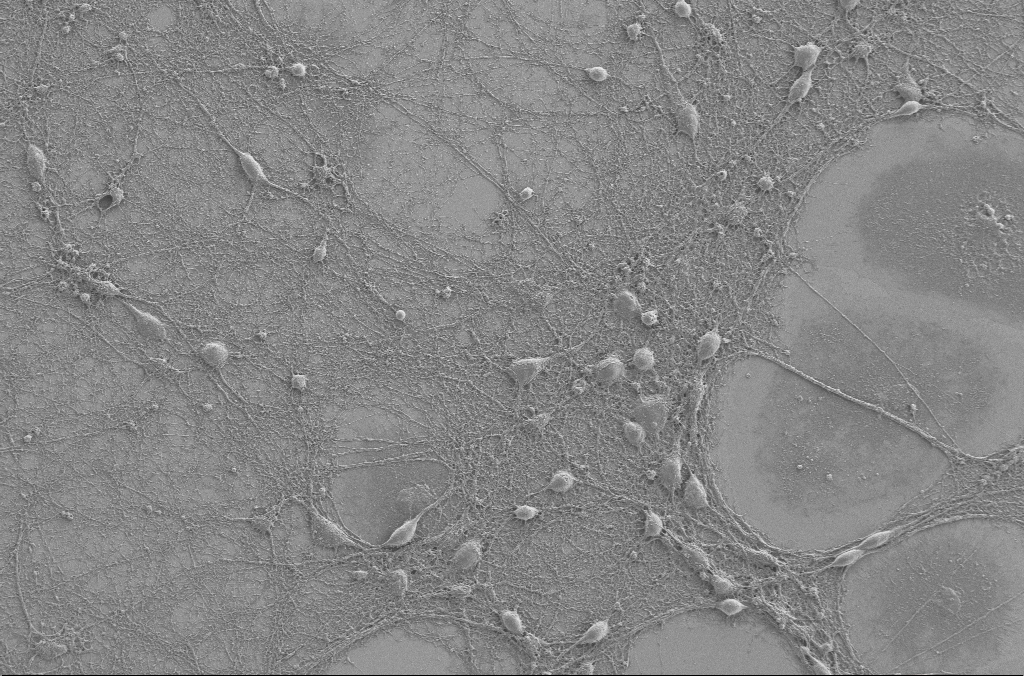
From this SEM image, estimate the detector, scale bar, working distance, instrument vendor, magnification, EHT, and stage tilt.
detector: SE2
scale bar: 20000 nm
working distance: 4 mm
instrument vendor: Zeiss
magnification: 1 K X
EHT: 2 kV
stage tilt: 0°